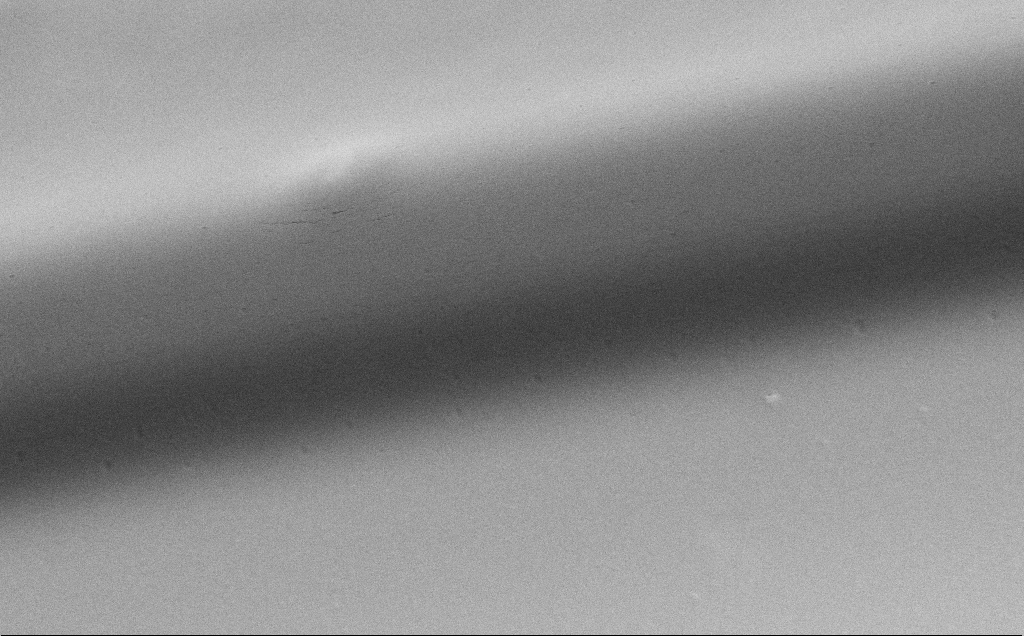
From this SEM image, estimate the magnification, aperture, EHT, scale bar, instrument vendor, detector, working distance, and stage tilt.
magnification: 37.92 K X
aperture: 30 µm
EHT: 5 kV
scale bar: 1000 nm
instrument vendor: Zeiss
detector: SE2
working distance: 11 mm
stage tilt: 30°